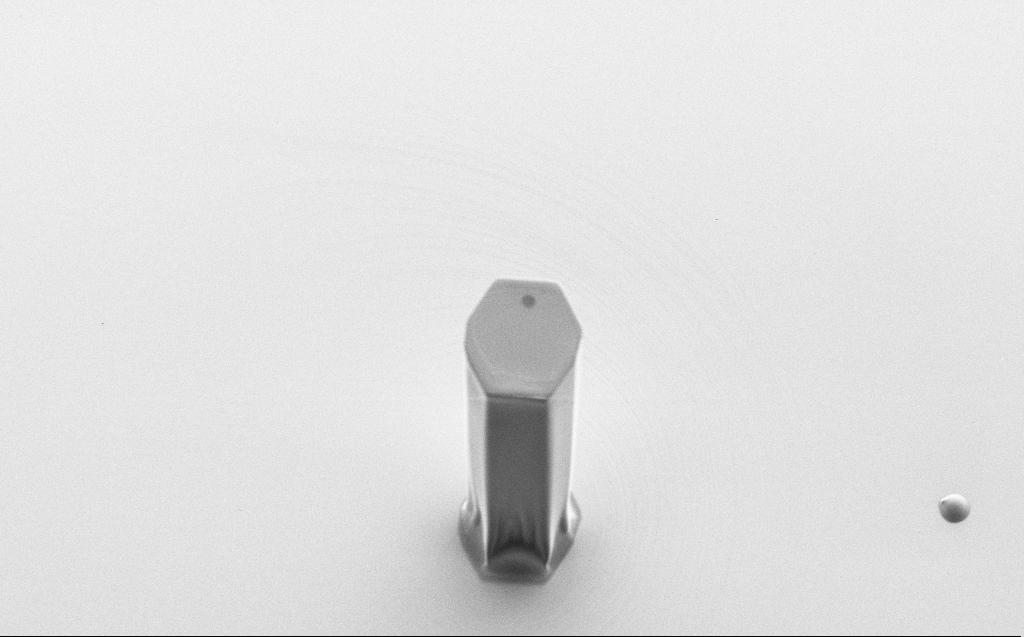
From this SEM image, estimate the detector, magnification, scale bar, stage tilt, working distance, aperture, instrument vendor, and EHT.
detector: SE2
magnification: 0.802 K X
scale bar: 20000 nm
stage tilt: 45°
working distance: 8 mm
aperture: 30 µm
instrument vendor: Zeiss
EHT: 1.7 kV